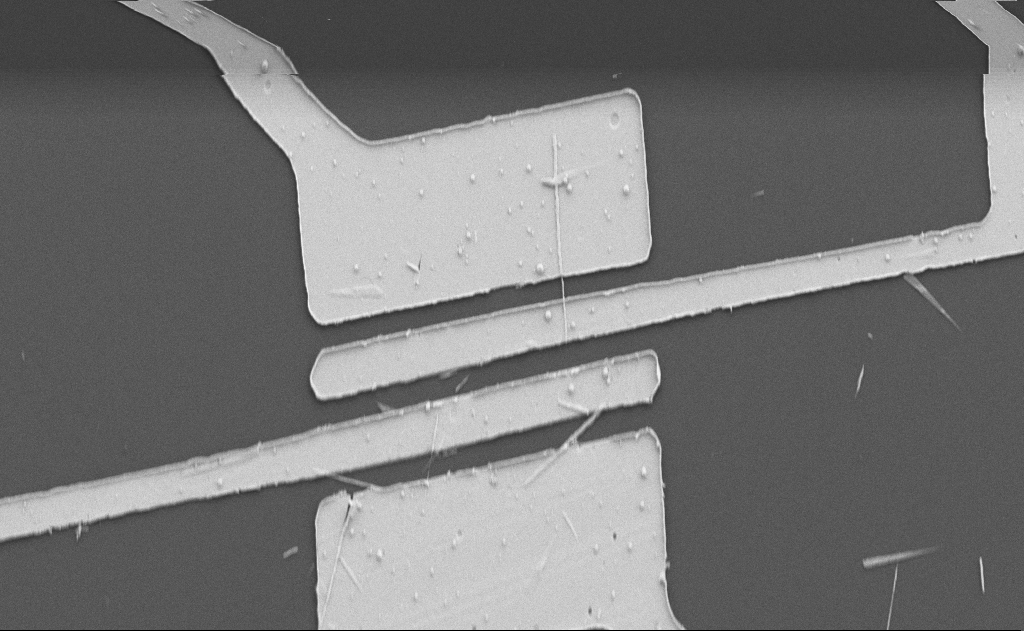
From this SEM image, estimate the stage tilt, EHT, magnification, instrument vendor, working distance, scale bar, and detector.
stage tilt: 0°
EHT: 5 kV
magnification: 4.37 K X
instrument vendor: Zeiss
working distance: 10 mm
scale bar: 10000 nm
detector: SE2